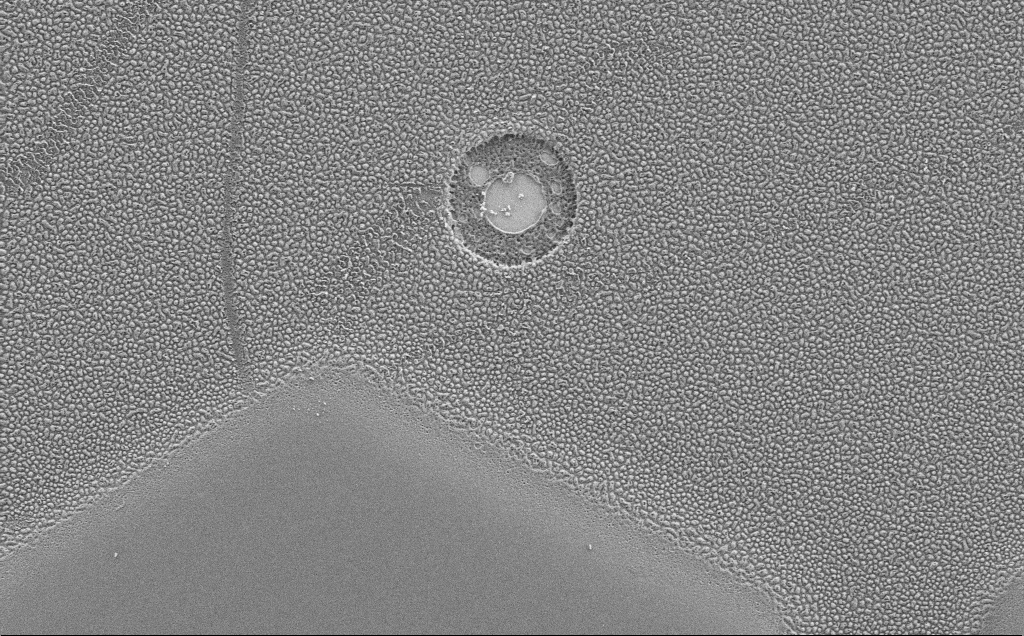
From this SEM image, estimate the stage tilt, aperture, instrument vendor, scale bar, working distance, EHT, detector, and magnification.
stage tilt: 0°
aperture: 30 µm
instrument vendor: Zeiss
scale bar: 10000 nm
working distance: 4 mm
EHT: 1.5 kV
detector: SE2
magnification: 6.83 K X